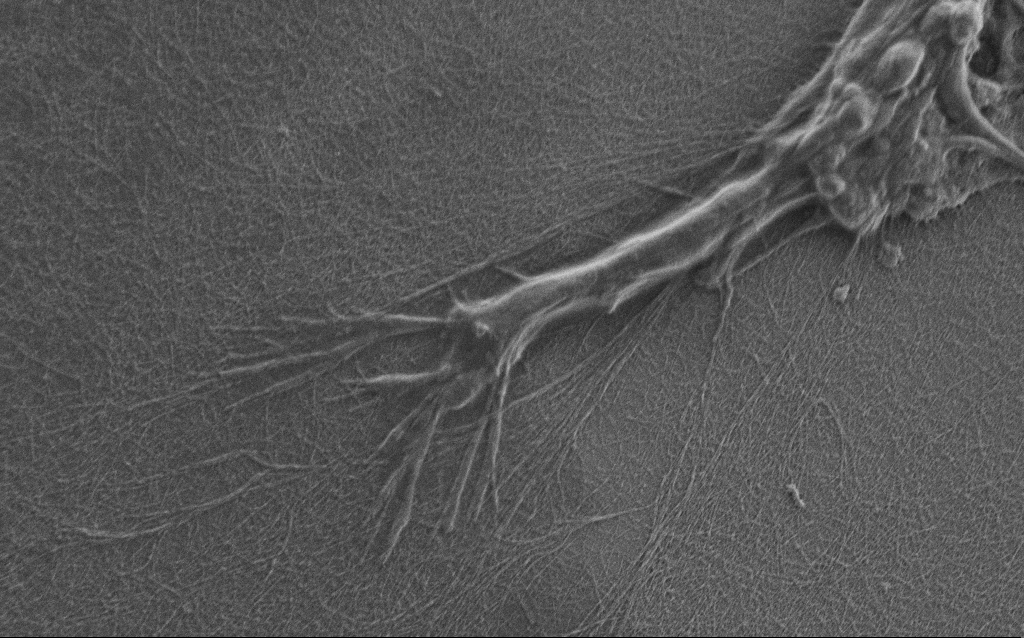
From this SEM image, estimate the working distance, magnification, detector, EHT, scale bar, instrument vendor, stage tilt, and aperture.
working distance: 6 mm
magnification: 10 K X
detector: SE2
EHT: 0.9 kV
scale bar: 2000 nm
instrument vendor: Zeiss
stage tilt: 0°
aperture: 30 µm